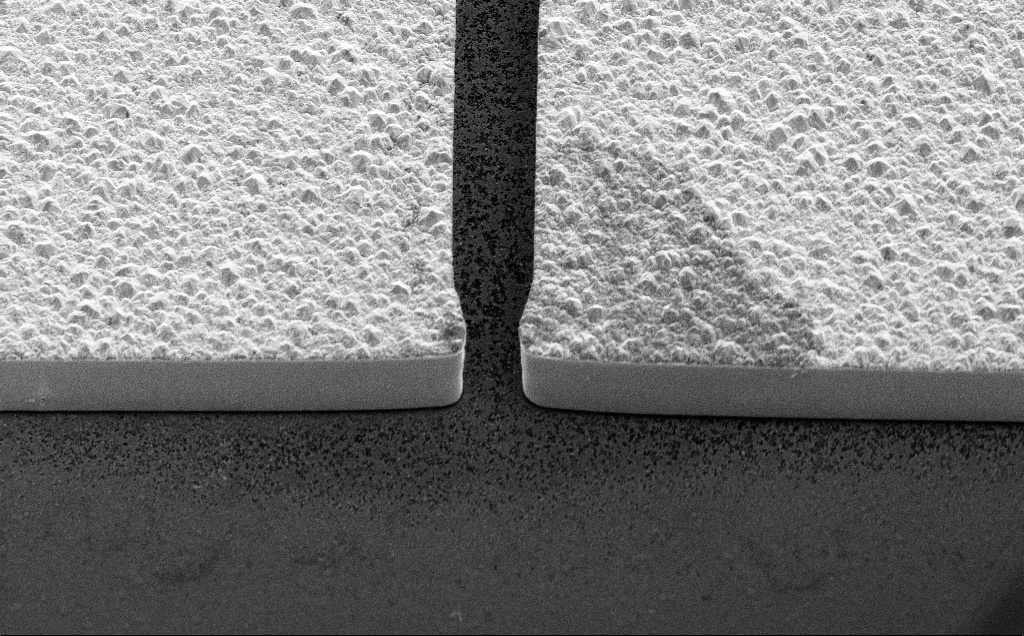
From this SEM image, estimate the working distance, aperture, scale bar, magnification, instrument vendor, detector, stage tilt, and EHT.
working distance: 17 mm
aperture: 30 µm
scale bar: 20000 nm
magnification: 3.22 K X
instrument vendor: Zeiss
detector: SE2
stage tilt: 45°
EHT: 10 kV